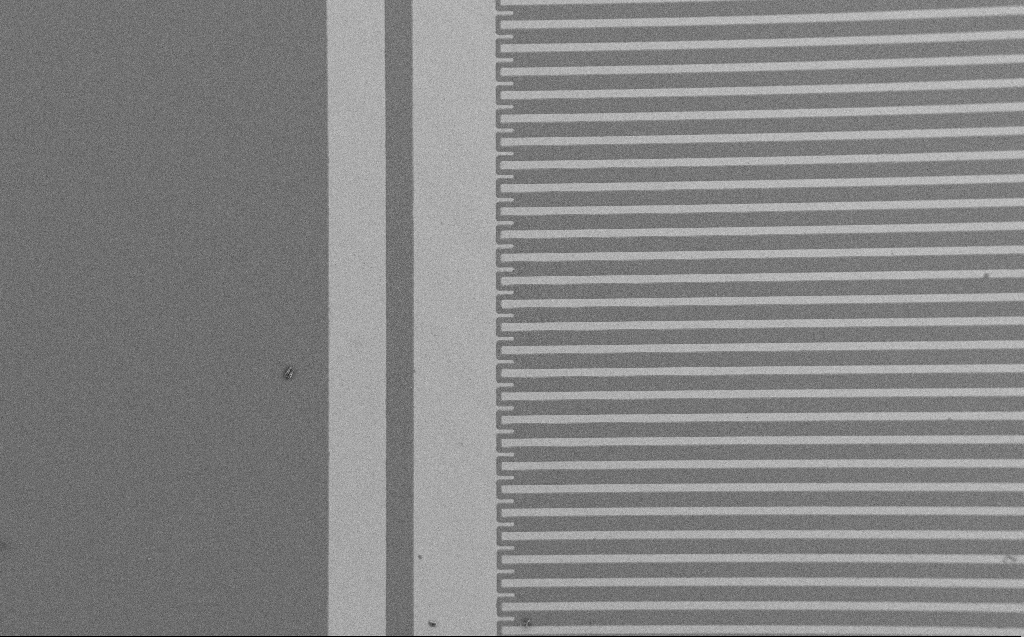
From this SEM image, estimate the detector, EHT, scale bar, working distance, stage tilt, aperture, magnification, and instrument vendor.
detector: SE2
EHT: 1.2 kV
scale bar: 100000 nm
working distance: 4 mm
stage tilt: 0°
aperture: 30 µm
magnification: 0.313 K X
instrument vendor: Zeiss